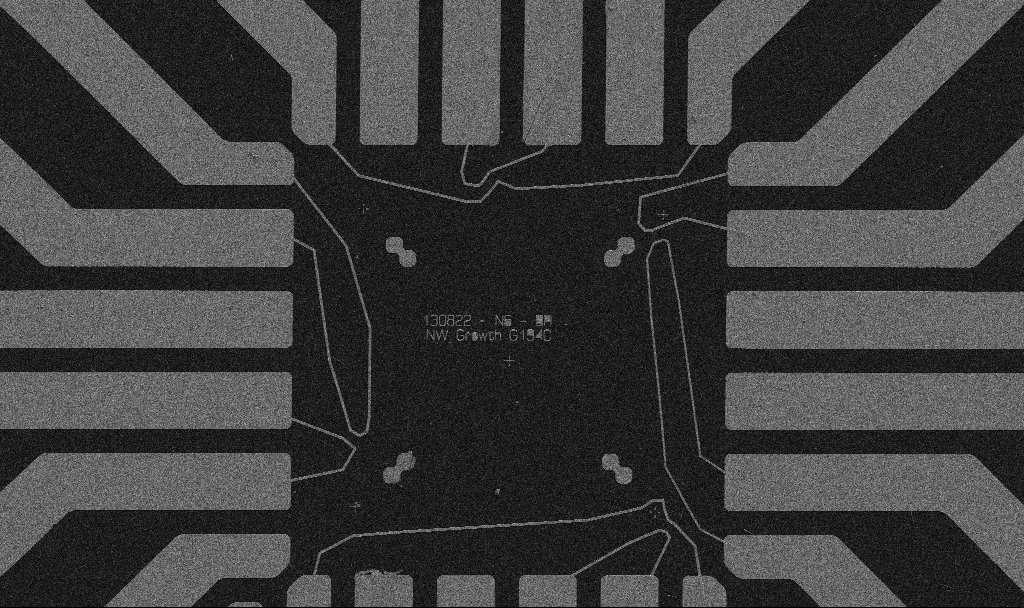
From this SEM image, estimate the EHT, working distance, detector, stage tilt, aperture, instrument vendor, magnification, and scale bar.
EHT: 5 kV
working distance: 10.7 mm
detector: SE2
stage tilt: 0°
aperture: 30 µm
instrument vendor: Zeiss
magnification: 1 K X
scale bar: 20000 nm